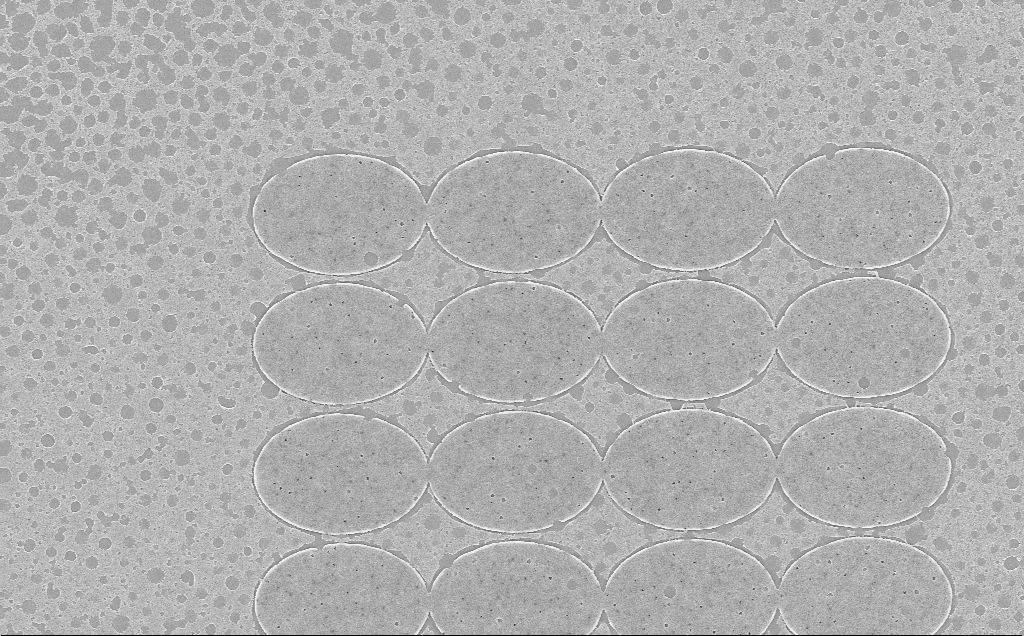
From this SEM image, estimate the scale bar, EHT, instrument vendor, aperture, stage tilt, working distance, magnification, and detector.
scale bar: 10000 nm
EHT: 5 kV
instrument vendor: Zeiss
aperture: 30 µm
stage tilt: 0°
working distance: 6 mm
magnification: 6.43 K X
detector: InLens